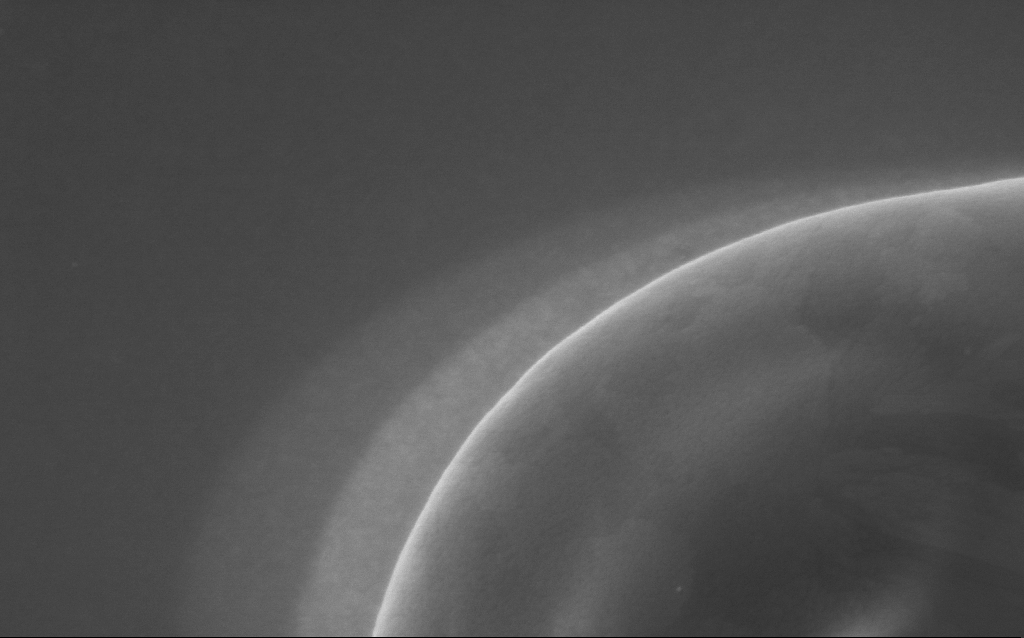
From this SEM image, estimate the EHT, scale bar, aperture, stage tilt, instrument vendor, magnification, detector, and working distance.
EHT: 5 kV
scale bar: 200 nm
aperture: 30 µm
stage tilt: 0°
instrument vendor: Zeiss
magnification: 99.78 K X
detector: InLens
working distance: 2 mm